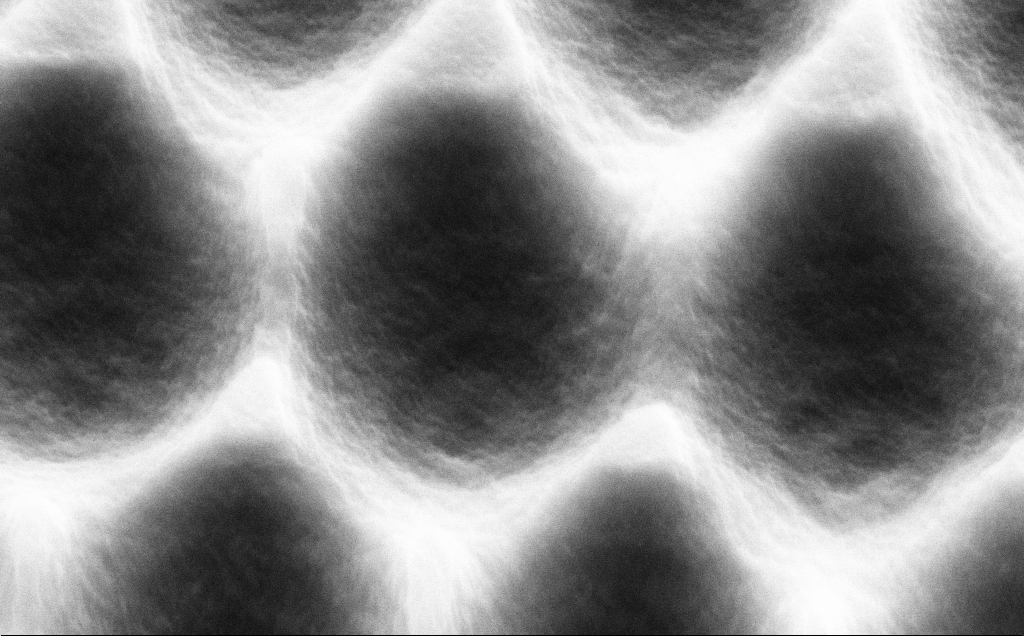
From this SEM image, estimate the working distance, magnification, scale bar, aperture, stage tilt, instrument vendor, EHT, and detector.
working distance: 10 mm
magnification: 72.18 K X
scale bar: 1000 nm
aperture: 30 µm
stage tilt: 45°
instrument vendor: Zeiss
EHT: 5 kV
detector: SE2